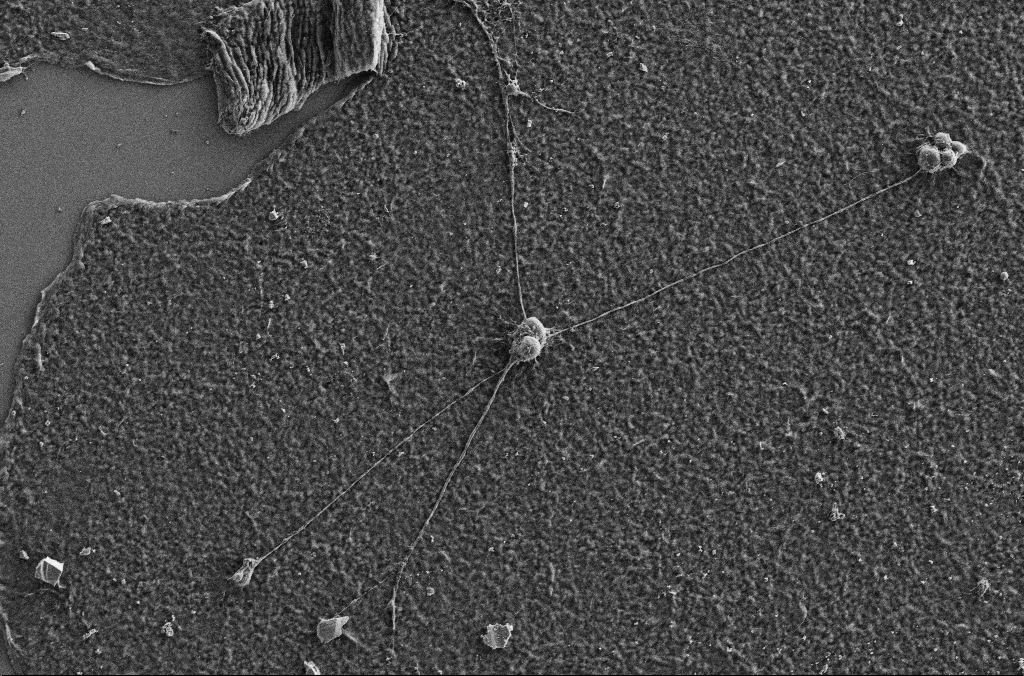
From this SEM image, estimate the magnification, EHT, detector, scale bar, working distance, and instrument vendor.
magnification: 1 K X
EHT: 5 kV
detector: SE2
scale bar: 20000 nm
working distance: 2.9 mm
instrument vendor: Zeiss